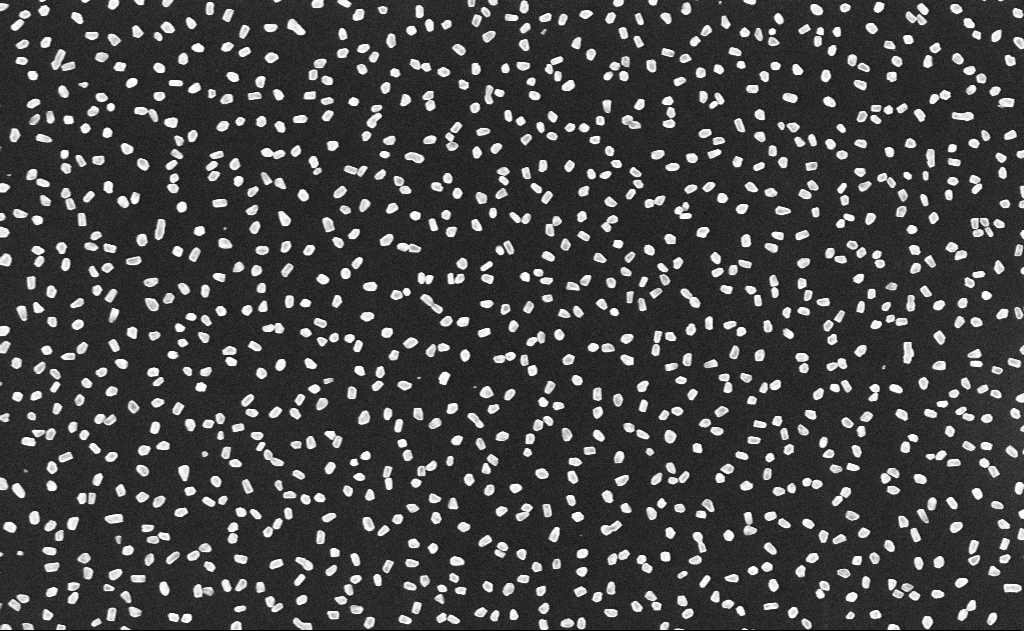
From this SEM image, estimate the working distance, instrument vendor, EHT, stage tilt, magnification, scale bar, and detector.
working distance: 11 mm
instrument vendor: Zeiss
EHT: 10 kV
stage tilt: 0°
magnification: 50 K X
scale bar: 1000 nm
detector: InLens